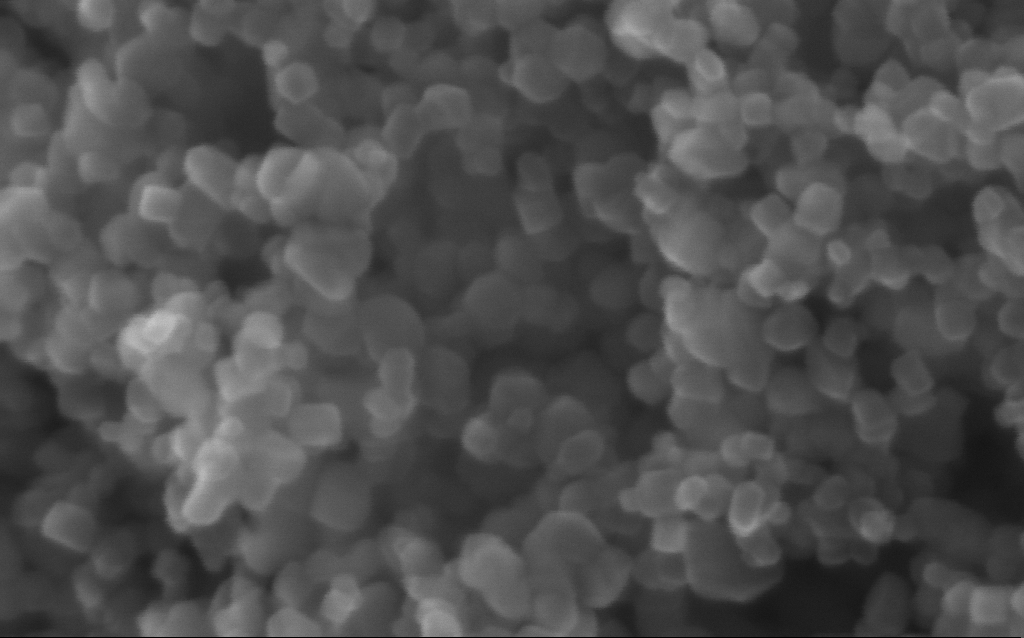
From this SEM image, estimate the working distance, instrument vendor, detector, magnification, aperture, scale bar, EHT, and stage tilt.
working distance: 2.8 mm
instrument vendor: Zeiss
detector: InLens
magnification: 716 K X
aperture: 30 µm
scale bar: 100 nm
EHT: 10 kV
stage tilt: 0°